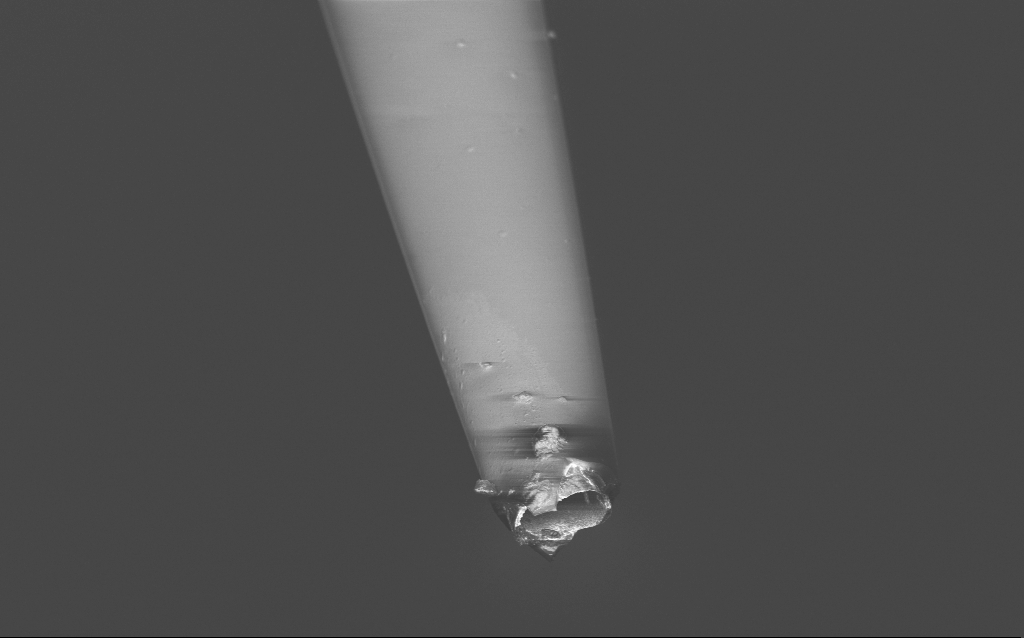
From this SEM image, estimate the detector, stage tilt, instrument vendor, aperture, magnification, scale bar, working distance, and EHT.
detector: InLens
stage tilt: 45°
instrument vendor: Zeiss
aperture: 30 µm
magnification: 5 K X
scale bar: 10000 nm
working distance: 6 mm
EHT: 2 kV